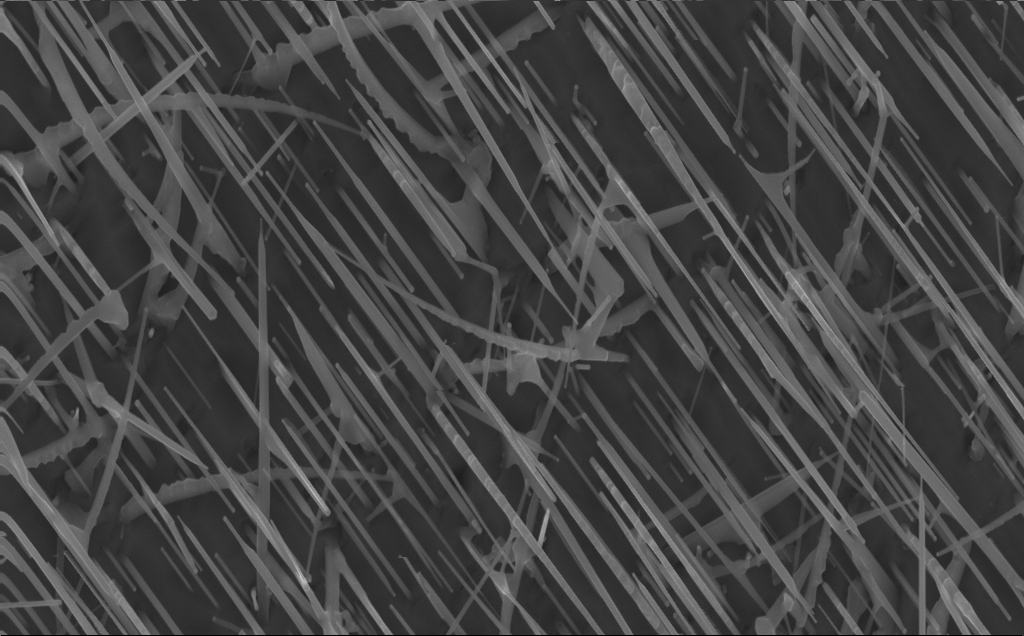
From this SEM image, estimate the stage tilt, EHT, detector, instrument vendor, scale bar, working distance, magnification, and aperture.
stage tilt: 0°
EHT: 10 kV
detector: InLens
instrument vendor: Zeiss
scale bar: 1000 nm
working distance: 4 mm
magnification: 40 K X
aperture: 30 µm